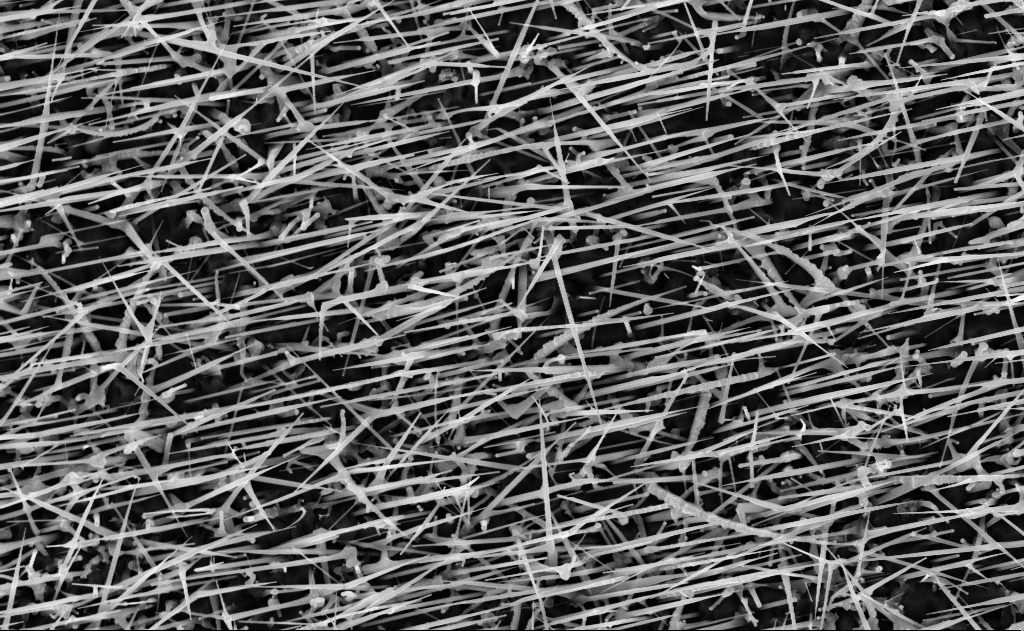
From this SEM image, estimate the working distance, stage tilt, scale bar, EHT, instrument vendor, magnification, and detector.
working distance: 15 mm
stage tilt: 0°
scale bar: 2000 nm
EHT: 10 kV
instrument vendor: Zeiss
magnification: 20 K X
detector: InLens